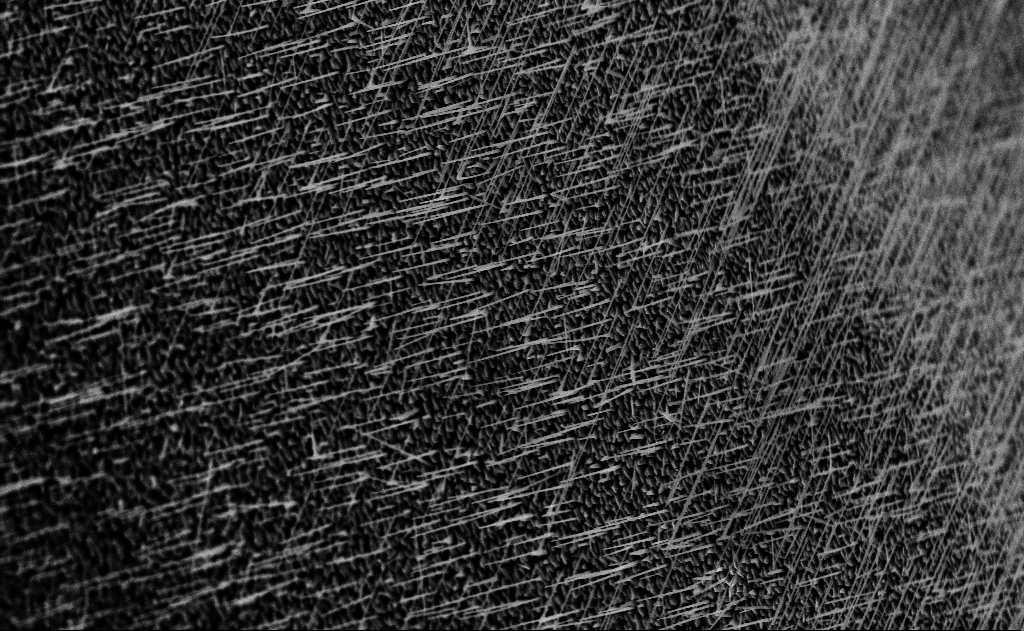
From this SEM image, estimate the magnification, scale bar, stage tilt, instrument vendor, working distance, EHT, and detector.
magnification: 10 K X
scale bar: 2000 nm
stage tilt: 0°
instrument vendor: Zeiss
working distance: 14 mm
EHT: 10 kV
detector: InLens